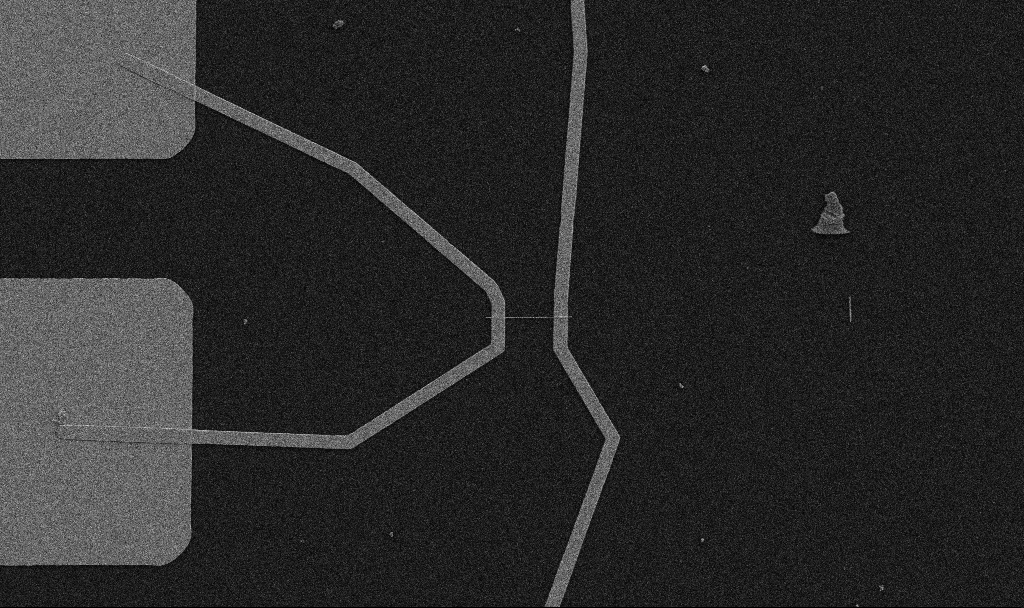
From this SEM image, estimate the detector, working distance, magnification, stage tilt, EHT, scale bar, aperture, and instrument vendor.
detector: SE2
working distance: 10.7 mm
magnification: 5 K X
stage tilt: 0°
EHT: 5 kV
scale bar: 10000 nm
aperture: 30 µm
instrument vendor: Zeiss